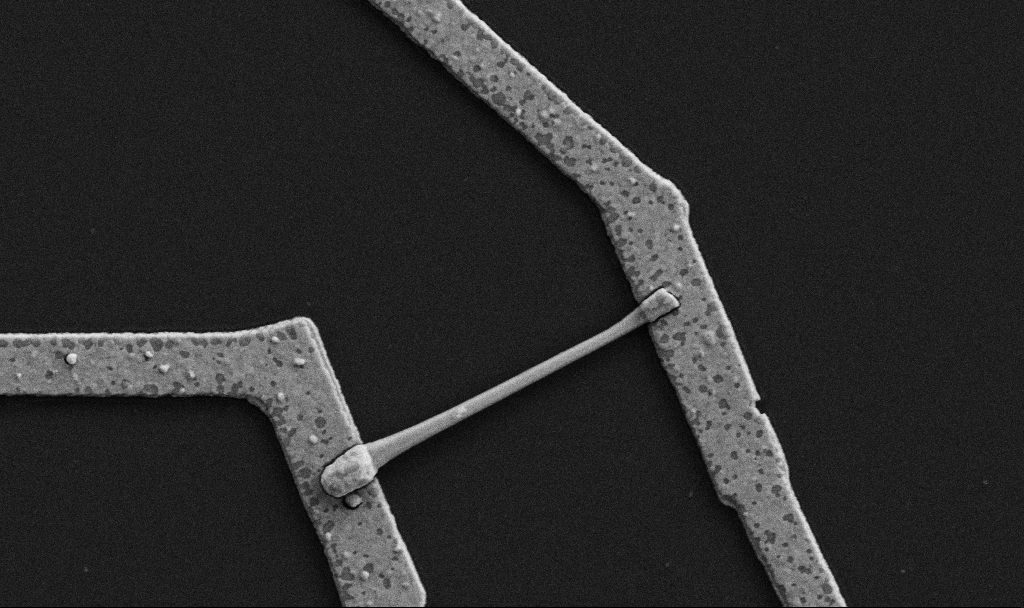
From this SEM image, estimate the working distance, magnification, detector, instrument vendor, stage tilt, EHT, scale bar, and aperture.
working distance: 8.7 mm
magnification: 30 K X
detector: SE2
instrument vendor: Zeiss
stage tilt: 0°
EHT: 5 kV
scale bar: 1000 nm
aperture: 30 µm